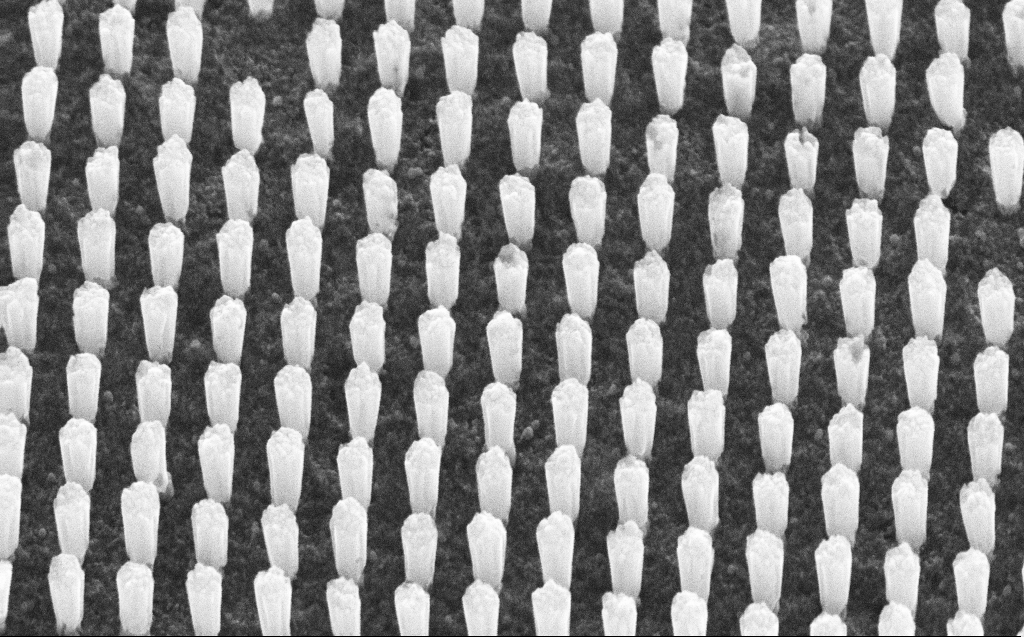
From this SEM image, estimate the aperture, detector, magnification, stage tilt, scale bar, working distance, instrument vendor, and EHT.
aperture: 30 µm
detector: SE2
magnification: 130.11 K X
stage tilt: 45°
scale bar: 200 nm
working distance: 5 mm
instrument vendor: Zeiss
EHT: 30 kV